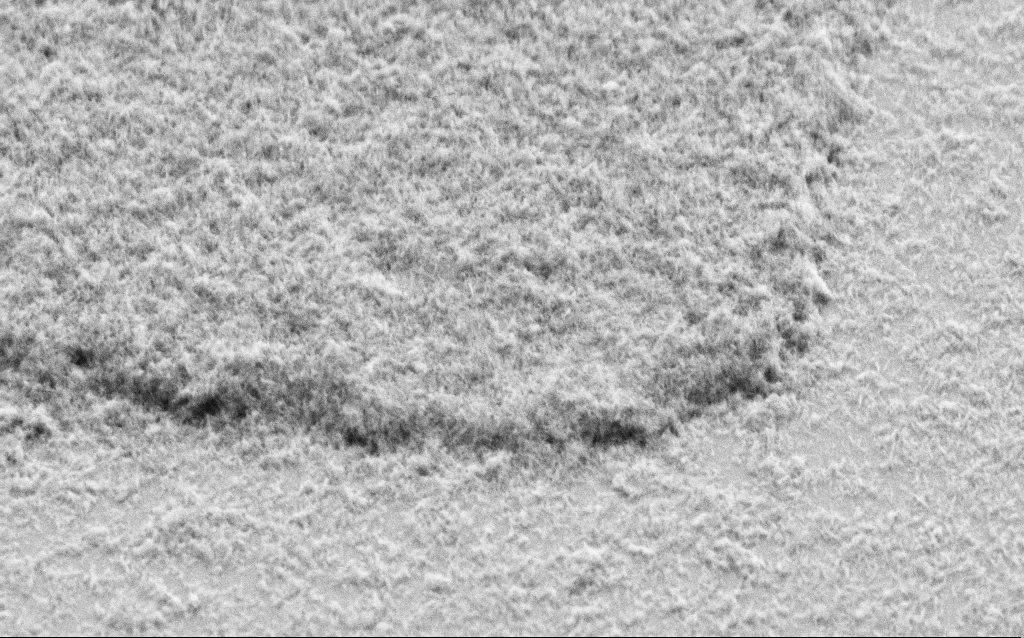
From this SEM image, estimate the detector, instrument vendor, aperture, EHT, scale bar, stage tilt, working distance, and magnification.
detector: SE2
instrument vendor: Zeiss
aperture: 30 µm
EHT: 2 kV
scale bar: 1000 nm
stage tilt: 45°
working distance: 5 mm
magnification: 37.82 K X